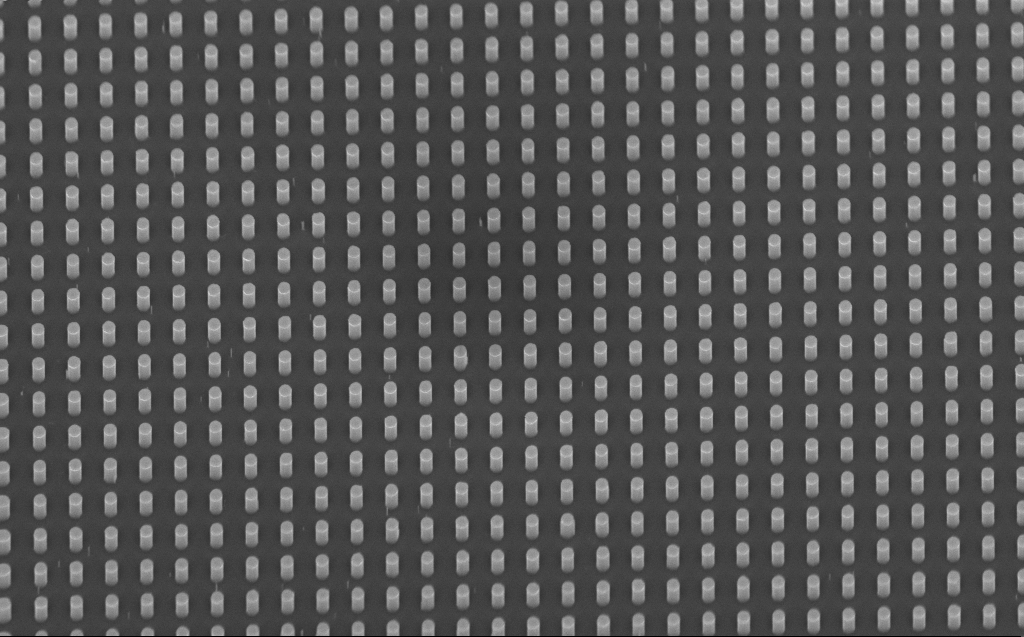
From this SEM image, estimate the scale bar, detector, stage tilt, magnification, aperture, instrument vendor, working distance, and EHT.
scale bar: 1000 nm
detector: InLens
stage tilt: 45°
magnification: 12.35 K X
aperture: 30 µm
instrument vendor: Zeiss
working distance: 6 mm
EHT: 5 kV